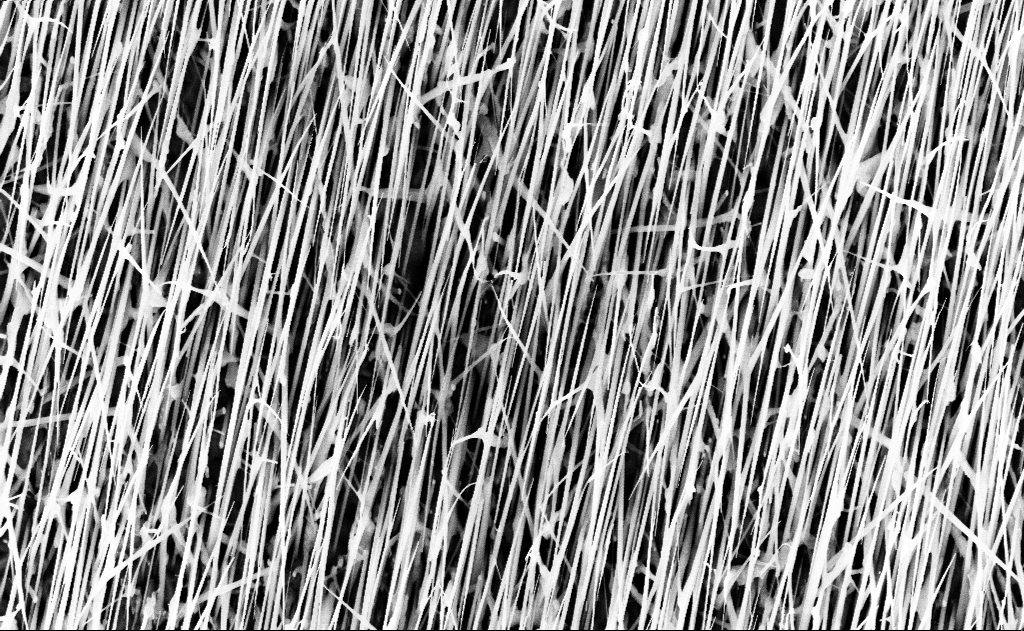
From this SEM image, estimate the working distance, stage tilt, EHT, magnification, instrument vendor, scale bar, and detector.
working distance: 16 mm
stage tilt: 0°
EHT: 10 kV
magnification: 20 K X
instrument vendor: Zeiss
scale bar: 2000 nm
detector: InLens